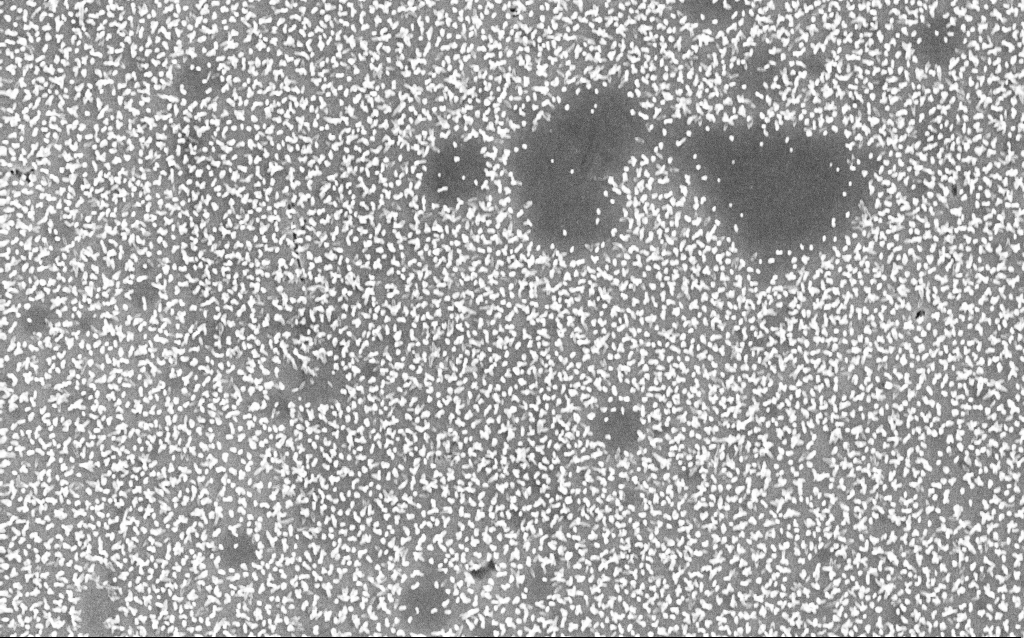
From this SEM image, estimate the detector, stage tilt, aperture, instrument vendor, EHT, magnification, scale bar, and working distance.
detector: InLens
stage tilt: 0°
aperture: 30 µm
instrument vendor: Zeiss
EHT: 6 kV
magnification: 10.27 K X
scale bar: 2000 nm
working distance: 5 mm